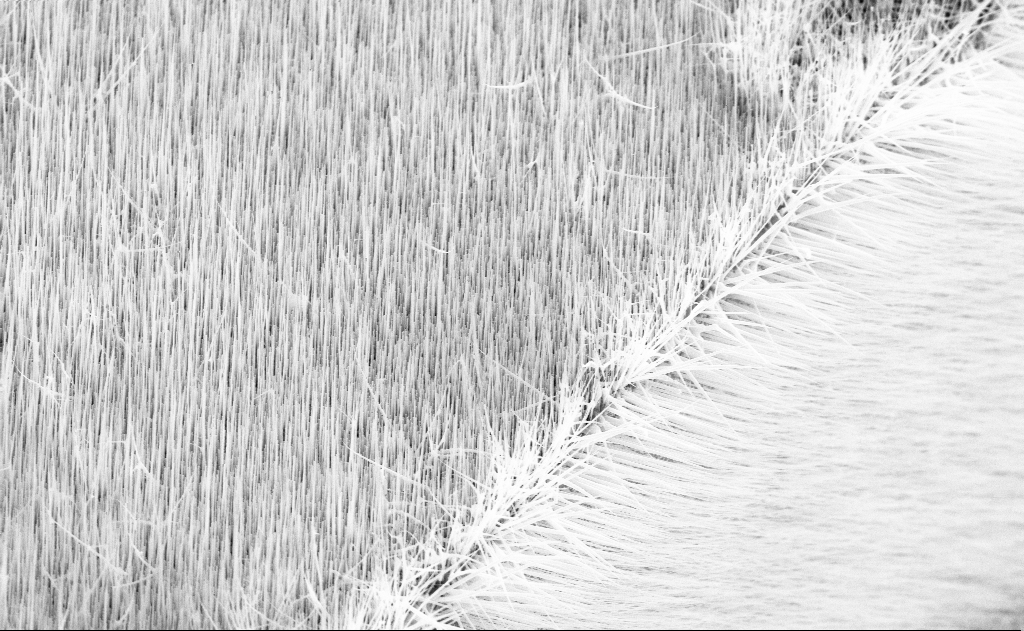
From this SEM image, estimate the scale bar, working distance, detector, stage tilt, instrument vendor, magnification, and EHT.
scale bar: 2000 nm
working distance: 16 mm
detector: SE2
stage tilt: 45°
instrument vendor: Zeiss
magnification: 10 K X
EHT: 10 kV